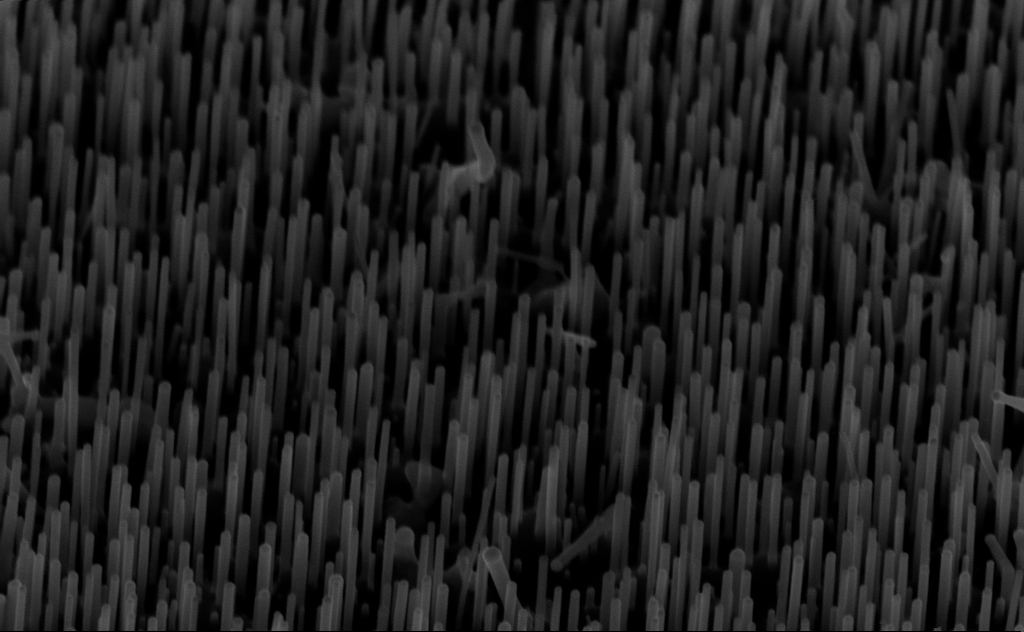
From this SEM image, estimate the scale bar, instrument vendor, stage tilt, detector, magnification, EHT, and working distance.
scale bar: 200 nm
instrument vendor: Zeiss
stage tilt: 45°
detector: InLens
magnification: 80 K X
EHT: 10 kV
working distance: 6 mm